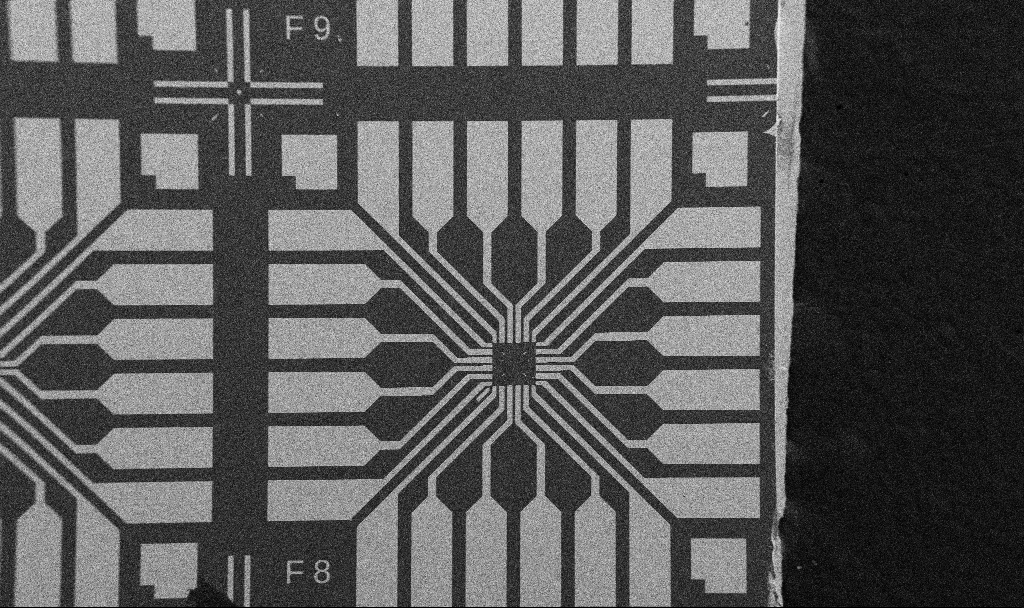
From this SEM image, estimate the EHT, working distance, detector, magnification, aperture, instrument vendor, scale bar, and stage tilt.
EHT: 5 kV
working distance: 10.7 mm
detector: SE2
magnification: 0.1 K X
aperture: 30 µm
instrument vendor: Zeiss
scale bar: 200000 nm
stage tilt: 0°